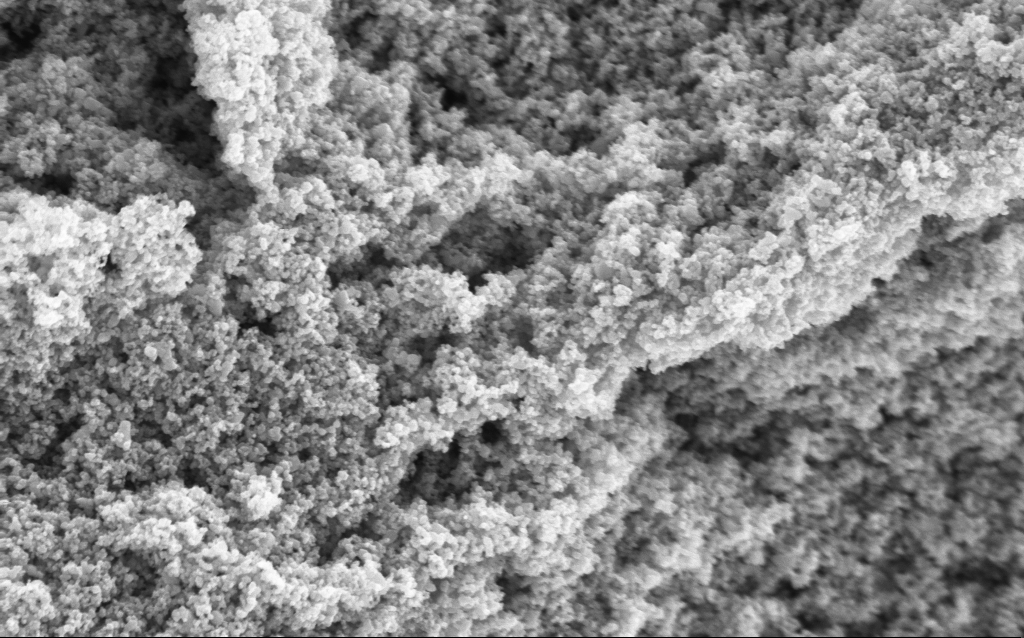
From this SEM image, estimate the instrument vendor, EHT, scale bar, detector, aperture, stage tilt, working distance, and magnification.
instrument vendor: Zeiss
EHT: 5 kV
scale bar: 1000 nm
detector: InLens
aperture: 30 µm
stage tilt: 0°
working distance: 4.4 mm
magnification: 68.66 K X